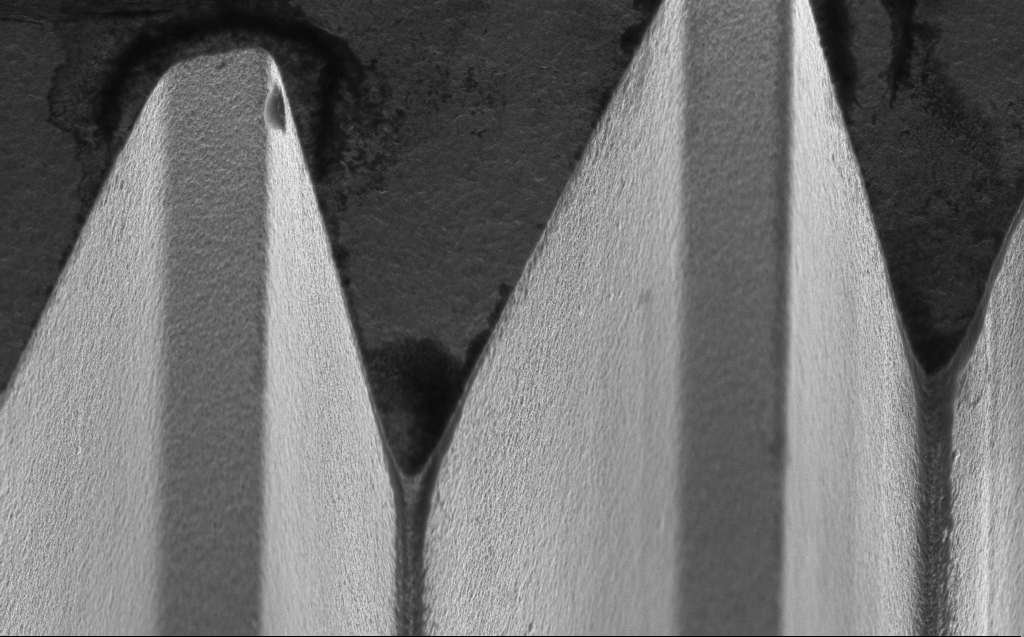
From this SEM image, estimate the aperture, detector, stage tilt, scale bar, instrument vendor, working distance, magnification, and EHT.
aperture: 30 µm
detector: InLens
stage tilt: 45°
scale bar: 1000 nm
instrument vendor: Zeiss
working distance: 5 mm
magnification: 17.62 K X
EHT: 5 kV